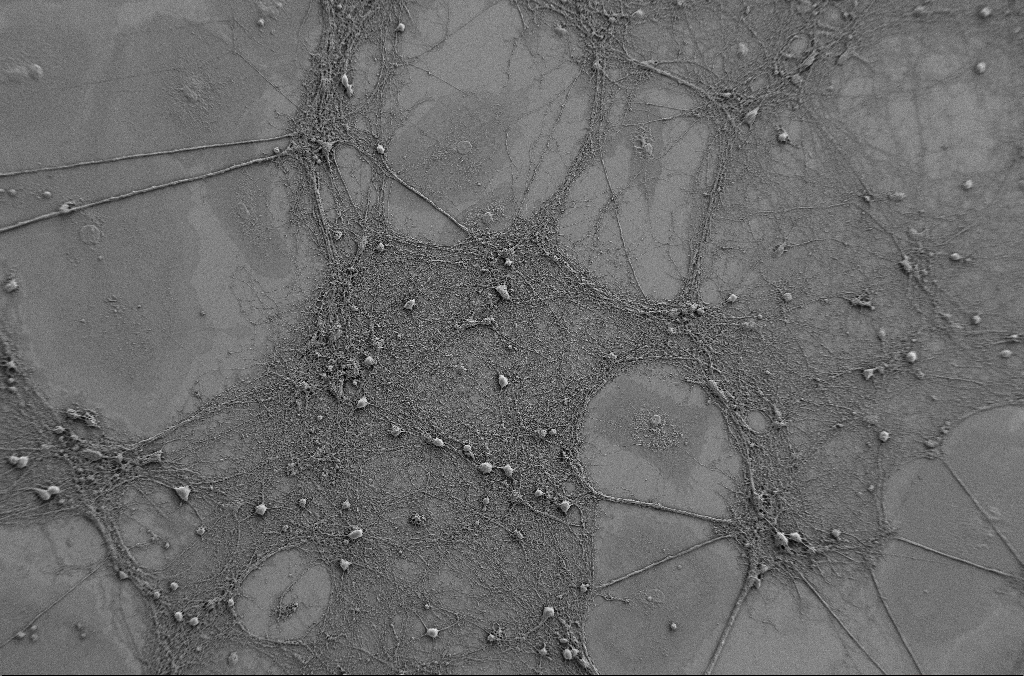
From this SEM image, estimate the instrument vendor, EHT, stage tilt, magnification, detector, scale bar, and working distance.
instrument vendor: Zeiss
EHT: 1 kV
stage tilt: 0°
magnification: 0.5 K X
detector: SE2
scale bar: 100000 nm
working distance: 4.1 mm